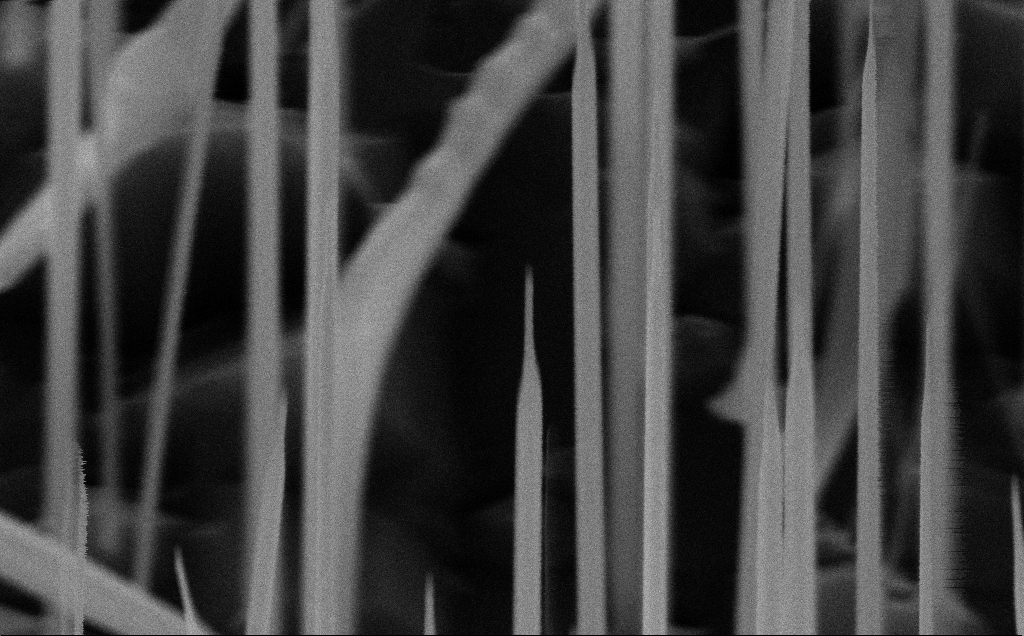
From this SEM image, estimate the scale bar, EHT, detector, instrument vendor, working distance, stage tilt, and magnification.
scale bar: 200 nm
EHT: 10 kV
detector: InLens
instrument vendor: Zeiss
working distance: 8 mm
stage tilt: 45°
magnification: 147.23 K X